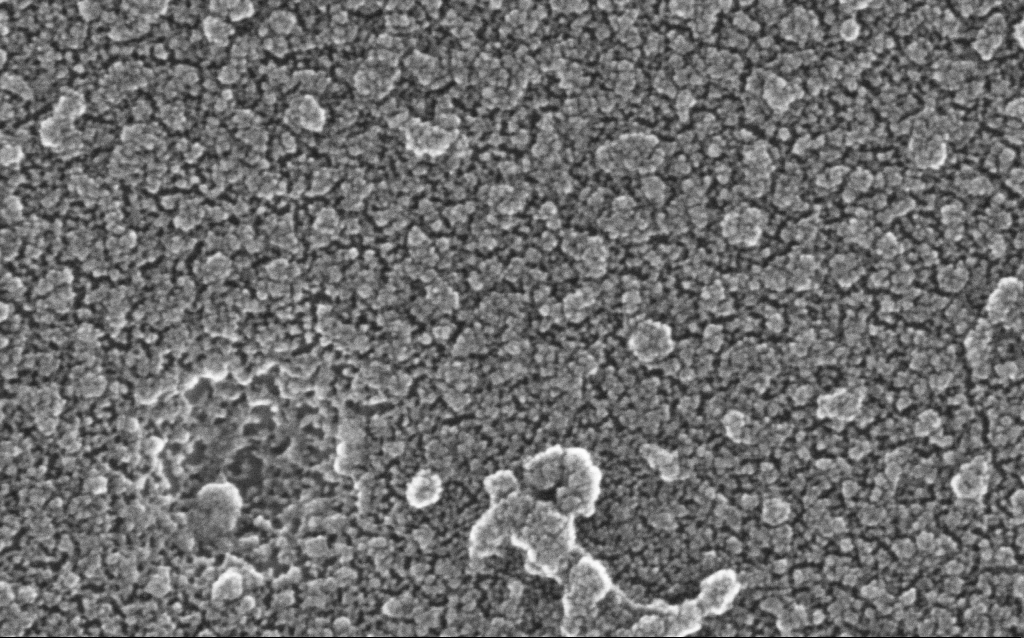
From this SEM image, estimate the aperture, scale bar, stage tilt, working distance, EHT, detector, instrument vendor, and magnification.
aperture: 30 µm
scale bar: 100 nm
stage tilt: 0°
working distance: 1.6 mm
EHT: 20 kV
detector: InLens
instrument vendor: Zeiss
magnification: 500 K X